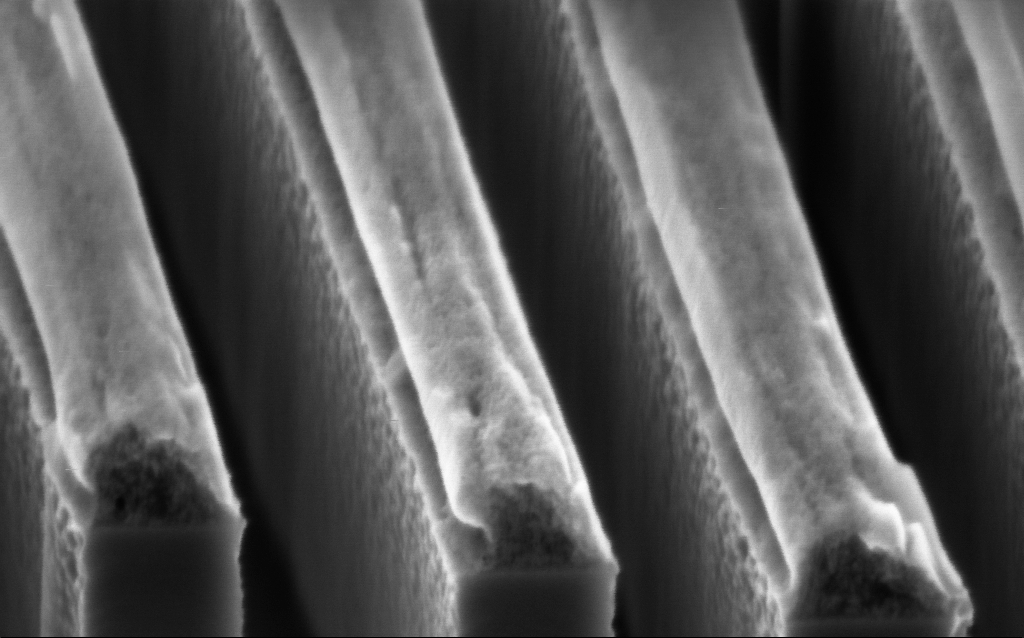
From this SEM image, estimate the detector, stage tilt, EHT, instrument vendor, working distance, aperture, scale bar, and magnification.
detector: InLens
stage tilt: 45°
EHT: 2 kV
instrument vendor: Zeiss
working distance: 3.6 mm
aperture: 30 µm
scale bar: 200 nm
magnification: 226.15 K X